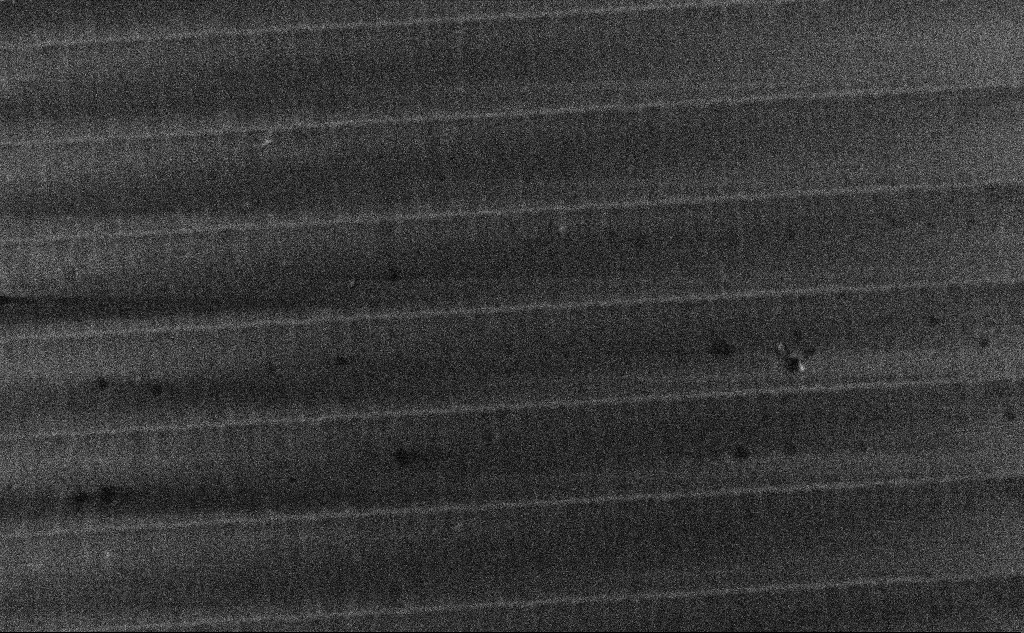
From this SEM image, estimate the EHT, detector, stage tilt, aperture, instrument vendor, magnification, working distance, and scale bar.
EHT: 3 kV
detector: InLens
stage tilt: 0°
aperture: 30 µm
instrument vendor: Zeiss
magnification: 17.95 K X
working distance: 6.3 mm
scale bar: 2000 nm